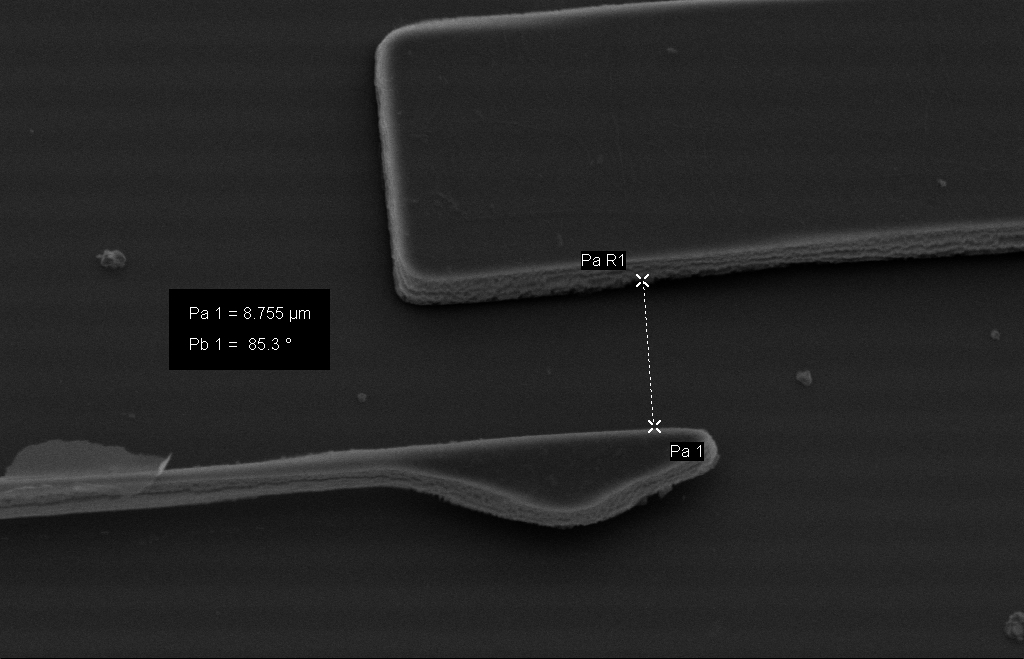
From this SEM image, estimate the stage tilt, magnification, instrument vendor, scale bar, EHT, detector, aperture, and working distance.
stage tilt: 23.3°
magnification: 6.14 K X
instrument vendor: Zeiss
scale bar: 2000 nm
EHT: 10 kV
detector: SE2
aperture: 30 µm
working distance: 20 mm